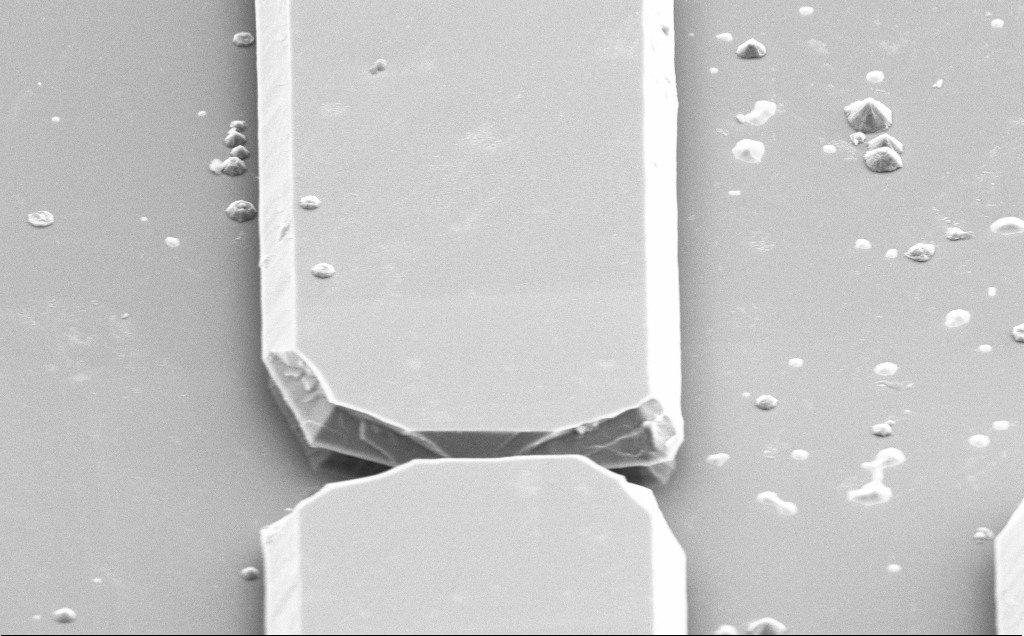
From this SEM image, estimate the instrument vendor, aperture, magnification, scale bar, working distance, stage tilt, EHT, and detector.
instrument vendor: Zeiss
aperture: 30 µm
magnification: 6.74 K X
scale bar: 10000 nm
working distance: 12 mm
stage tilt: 50°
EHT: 5 kV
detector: SE2